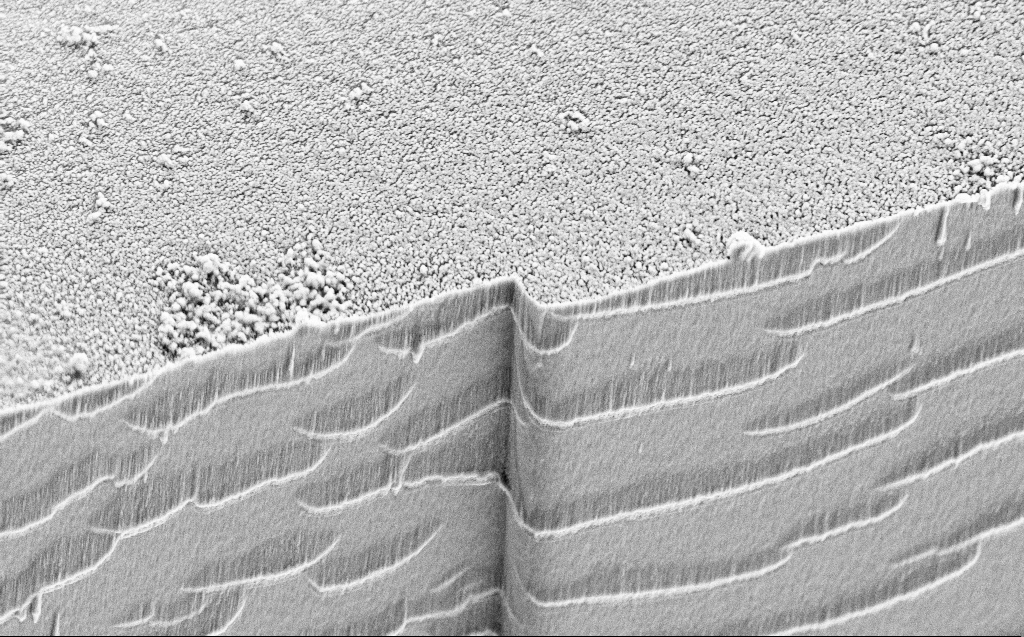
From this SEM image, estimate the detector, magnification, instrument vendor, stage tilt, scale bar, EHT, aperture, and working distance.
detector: SE2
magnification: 10.27 K X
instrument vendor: Zeiss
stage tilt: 45°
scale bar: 2000 nm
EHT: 5 kV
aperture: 30 µm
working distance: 4 mm